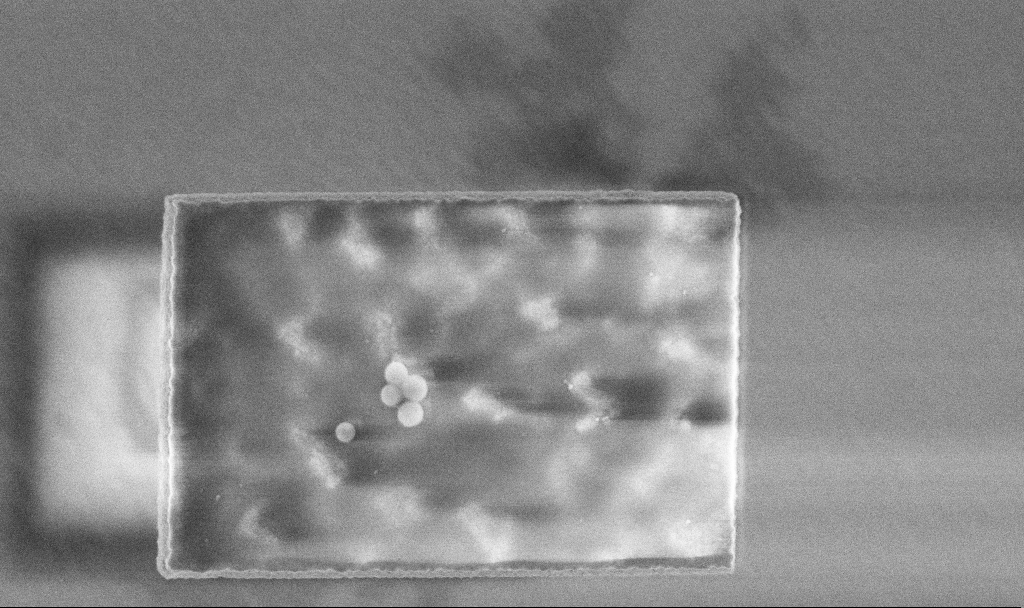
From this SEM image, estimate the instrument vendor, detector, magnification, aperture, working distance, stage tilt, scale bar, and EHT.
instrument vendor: Zeiss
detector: InLens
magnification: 46.46 K X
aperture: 30 µm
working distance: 3.3 mm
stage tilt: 0°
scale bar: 1000 nm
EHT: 3 kV